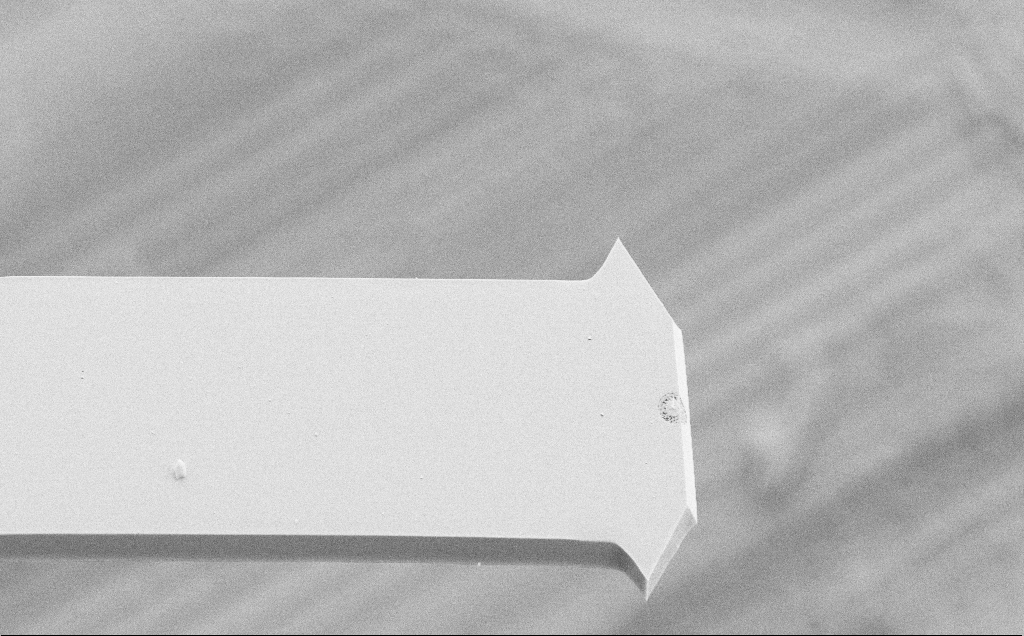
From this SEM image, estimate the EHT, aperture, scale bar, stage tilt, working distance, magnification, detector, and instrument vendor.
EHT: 10 kV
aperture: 30 µm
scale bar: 20000 nm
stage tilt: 44.2°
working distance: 3 mm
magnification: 2.82 K X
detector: SE2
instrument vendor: Zeiss